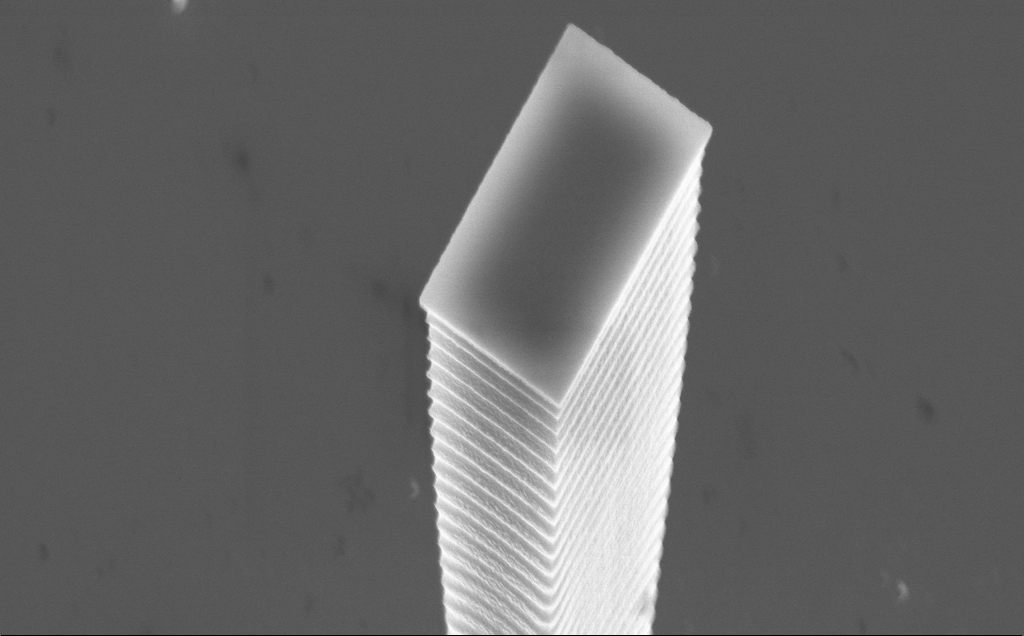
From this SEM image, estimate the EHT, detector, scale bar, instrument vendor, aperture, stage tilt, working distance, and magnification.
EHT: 10 kV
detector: InLens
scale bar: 1000 nm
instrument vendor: Zeiss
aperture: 30 µm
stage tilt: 36.9°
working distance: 4 mm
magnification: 21.73 K X